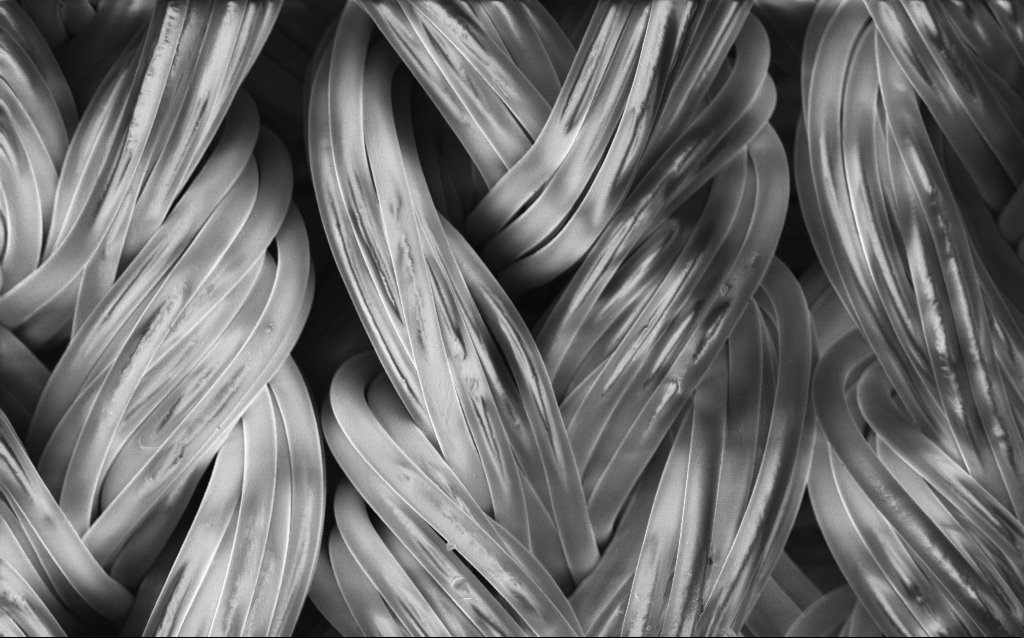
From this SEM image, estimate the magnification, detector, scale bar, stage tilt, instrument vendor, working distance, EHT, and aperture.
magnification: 0.523 K X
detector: InLens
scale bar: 100000 nm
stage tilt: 0°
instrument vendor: Zeiss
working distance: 4 mm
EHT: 2 kV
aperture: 30 µm